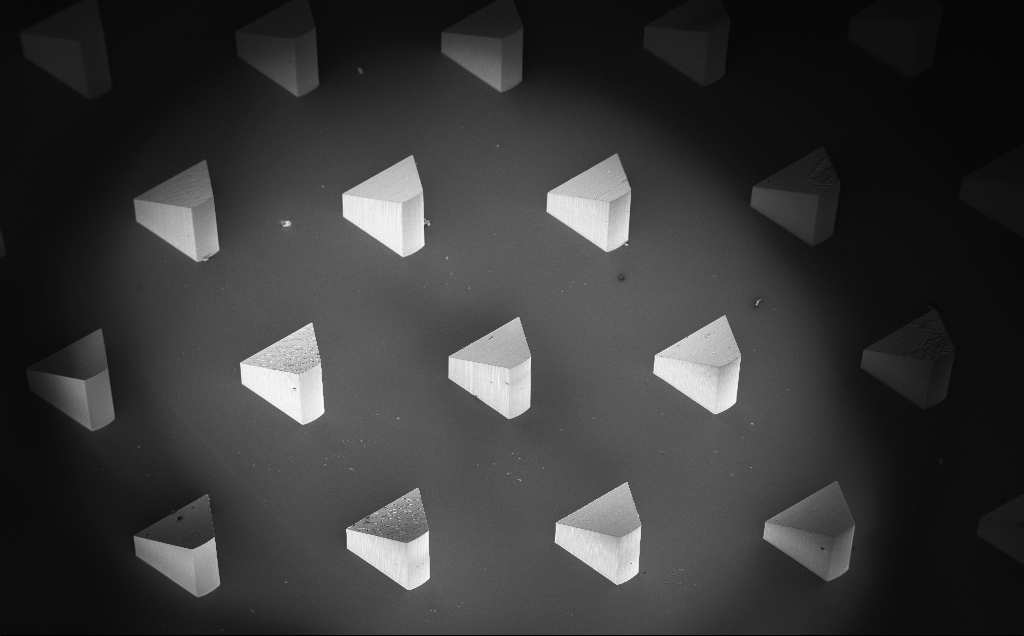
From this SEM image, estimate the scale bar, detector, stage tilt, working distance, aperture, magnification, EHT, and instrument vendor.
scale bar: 200000 nm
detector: InLens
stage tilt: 20°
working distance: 9 mm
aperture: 30 µm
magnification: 0.077 K X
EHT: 10 kV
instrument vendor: Zeiss